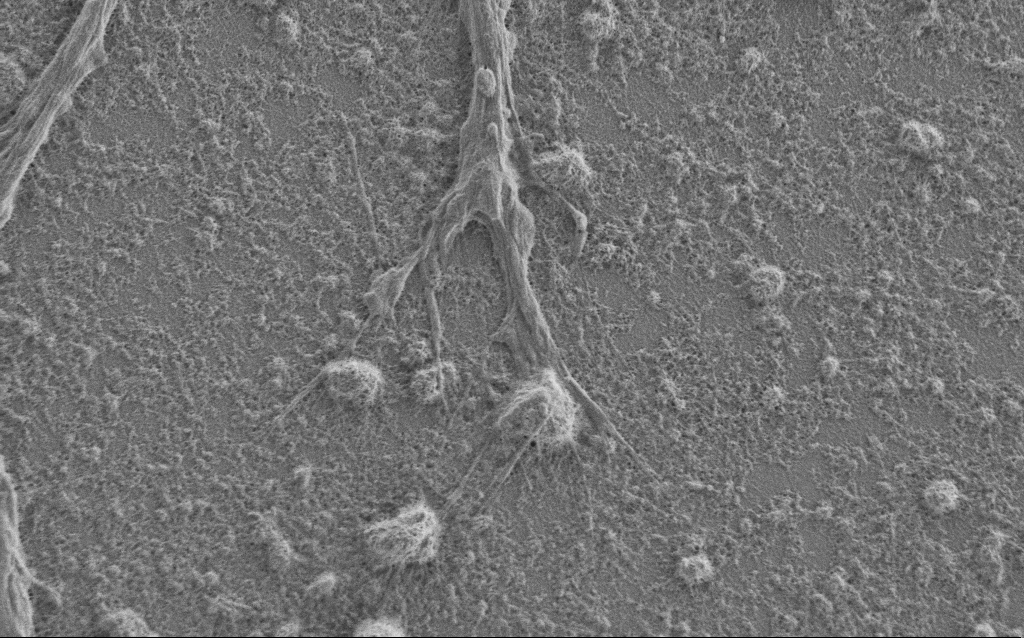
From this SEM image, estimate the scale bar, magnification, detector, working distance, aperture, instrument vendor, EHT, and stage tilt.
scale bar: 2000 nm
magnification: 7.5 K X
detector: SE2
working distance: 6 mm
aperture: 30 µm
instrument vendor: Zeiss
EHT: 1 kV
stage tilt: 0°